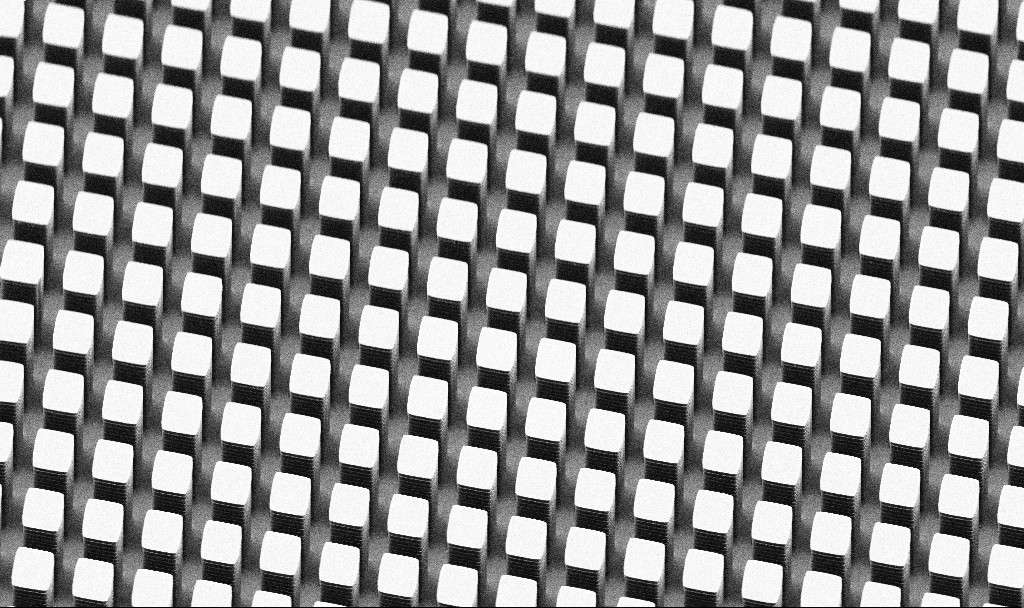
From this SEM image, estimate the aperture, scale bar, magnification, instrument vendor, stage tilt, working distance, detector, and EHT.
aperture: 30 µm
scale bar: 10000 nm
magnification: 3.69 K X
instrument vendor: Zeiss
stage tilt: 45°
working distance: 7.5 mm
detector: SE2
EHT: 5 kV